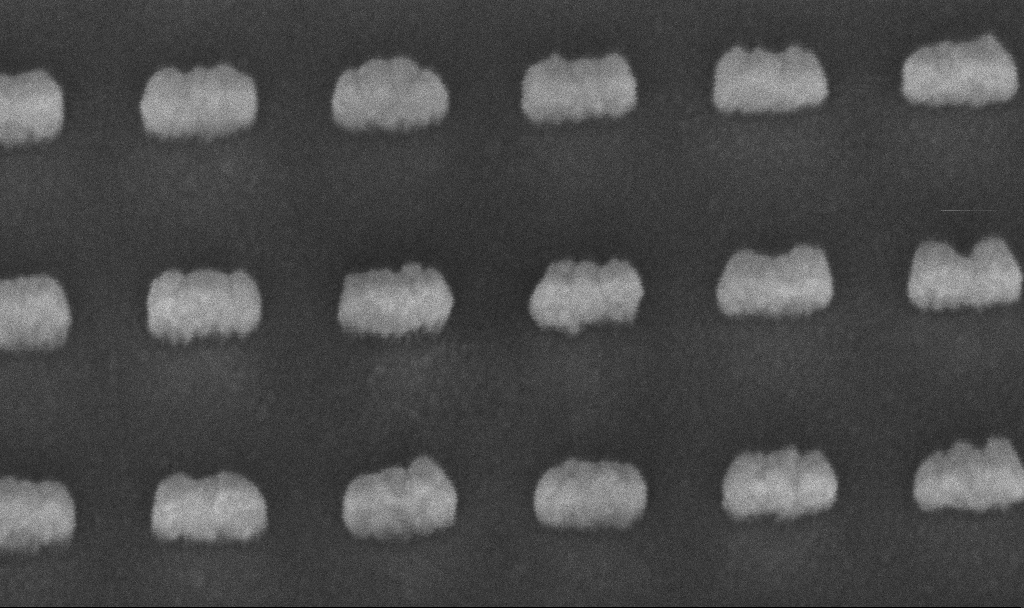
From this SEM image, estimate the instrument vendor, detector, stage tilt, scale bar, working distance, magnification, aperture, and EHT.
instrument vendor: Zeiss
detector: InLens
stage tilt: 45°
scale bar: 200 nm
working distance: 6.7 mm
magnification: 303.1 K X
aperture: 30 µm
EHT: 5 kV